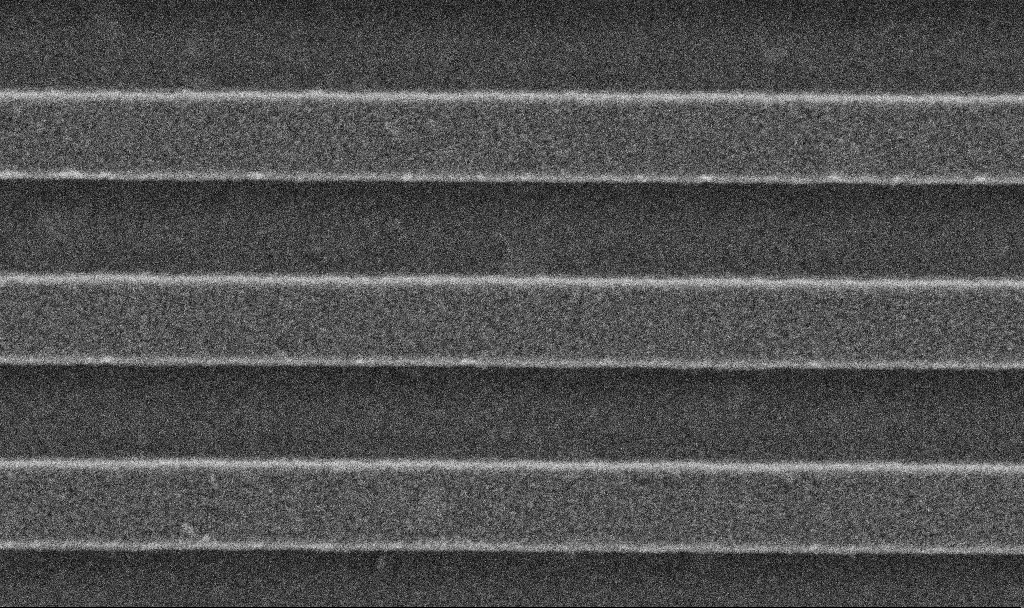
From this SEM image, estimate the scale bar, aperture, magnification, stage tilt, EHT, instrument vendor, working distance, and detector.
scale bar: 200 nm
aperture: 30 µm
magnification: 75.77 K X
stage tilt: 0°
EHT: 5 kV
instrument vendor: Zeiss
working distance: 2.9 mm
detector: SE2